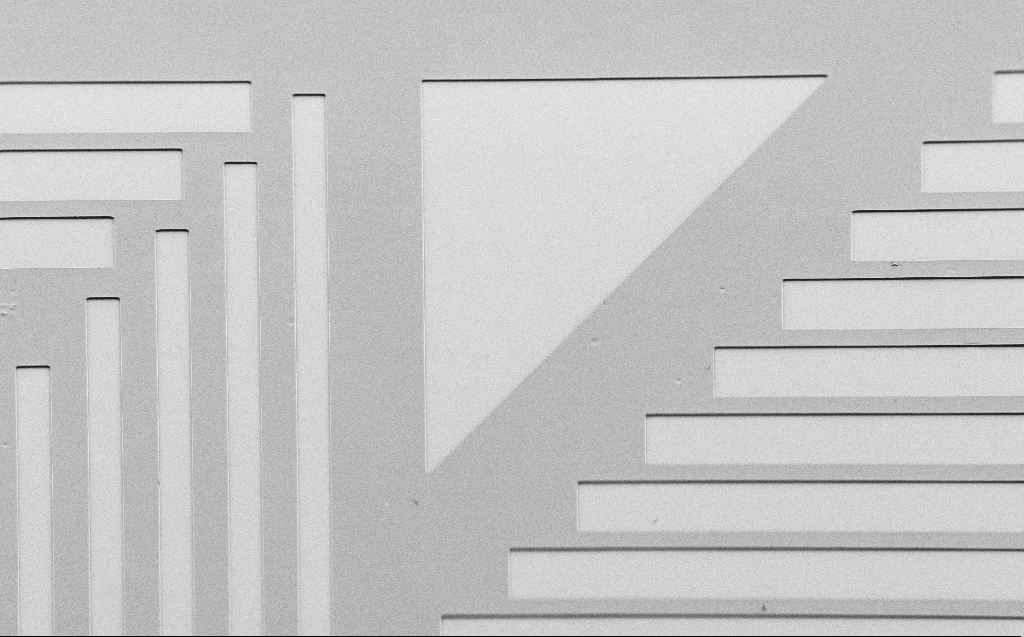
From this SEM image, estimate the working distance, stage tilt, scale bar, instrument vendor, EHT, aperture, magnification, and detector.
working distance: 6 mm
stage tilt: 45°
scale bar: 200000 nm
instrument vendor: Zeiss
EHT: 1.7 kV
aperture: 30 µm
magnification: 0.338 K X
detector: SE2